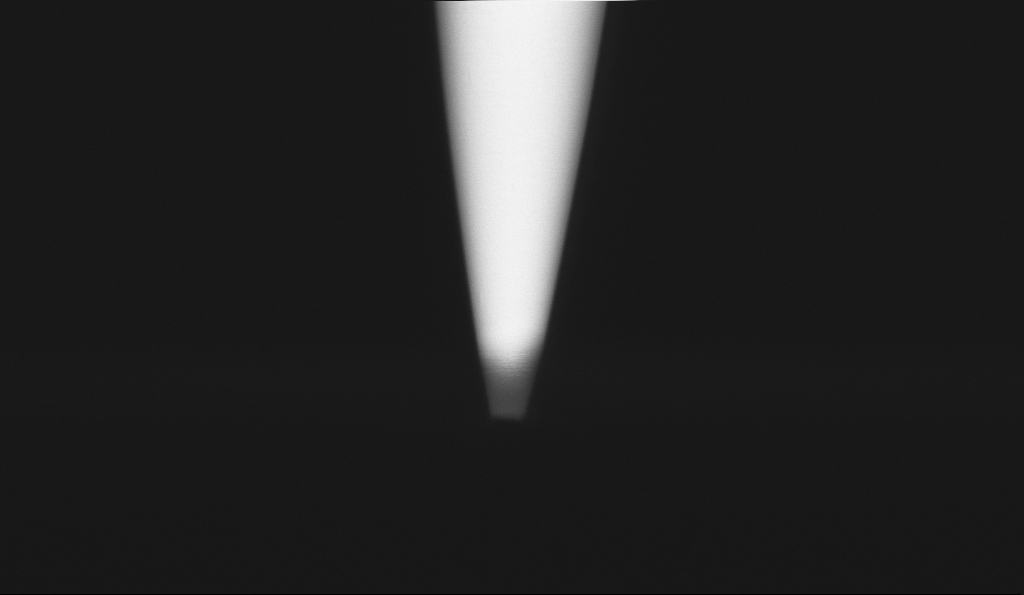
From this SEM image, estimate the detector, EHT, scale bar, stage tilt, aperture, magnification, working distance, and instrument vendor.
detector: InLens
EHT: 1 kV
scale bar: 1000 nm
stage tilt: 0°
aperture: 30 µm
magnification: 50 K X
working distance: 5.5 mm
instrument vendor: Zeiss